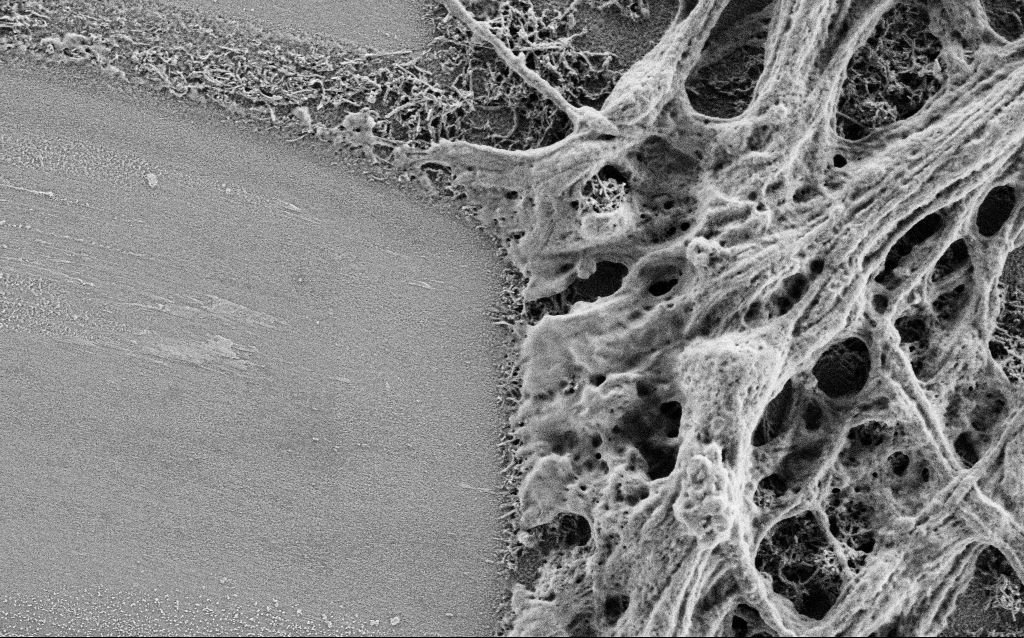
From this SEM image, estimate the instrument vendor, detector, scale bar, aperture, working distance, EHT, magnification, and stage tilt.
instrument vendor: Zeiss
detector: SE2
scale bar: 1000 nm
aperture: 30 µm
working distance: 4 mm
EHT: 1 kV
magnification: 25 K X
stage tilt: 0°